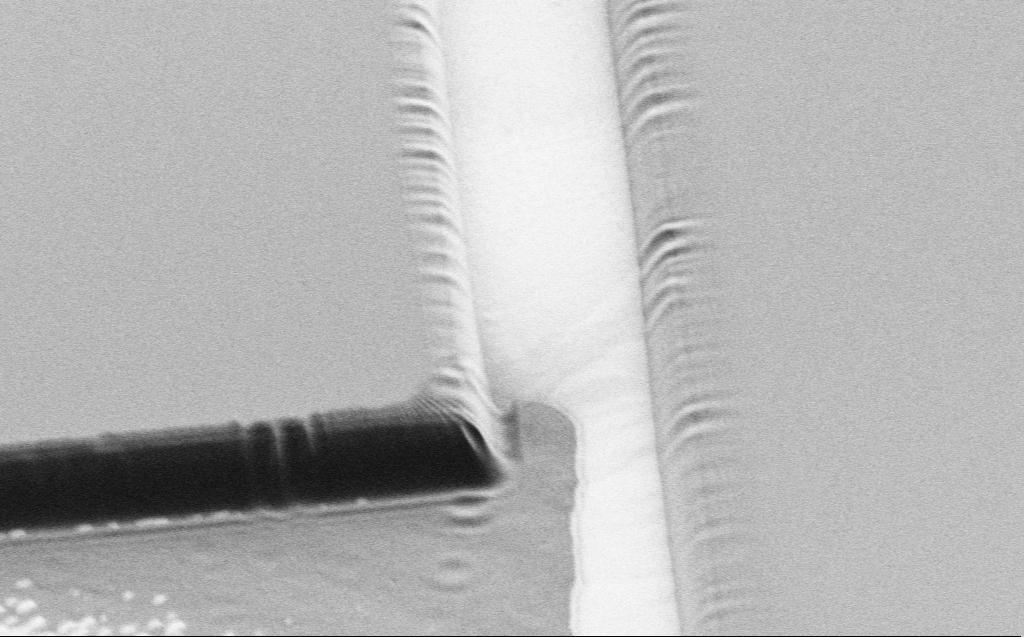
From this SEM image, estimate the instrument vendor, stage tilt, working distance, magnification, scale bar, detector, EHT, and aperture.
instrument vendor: Zeiss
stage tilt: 45°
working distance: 7 mm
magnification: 2.98 K X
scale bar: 20000 nm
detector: SE2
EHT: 1 kV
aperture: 30 µm